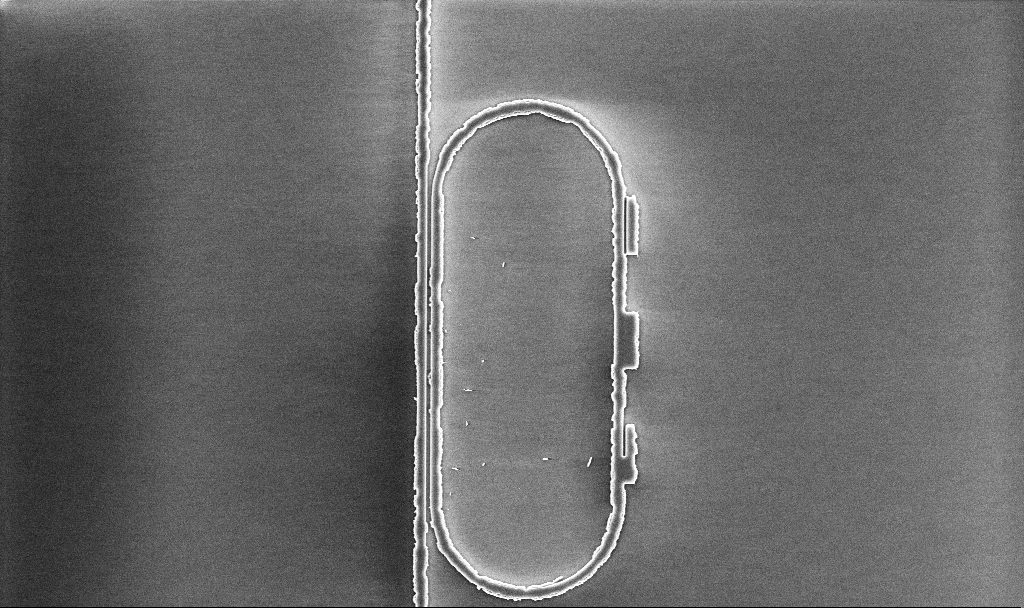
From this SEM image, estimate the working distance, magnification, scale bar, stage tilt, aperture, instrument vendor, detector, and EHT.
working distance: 10.1 mm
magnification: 7.13 K X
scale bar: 10000 nm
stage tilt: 0°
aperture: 30 µm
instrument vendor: Zeiss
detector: InLens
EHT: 5 kV